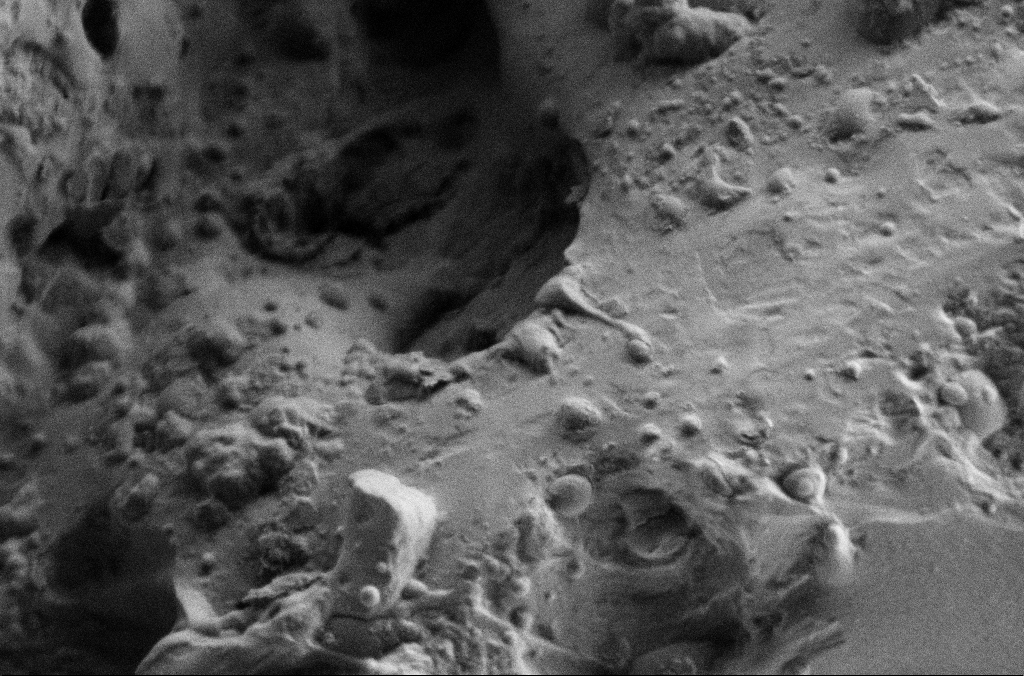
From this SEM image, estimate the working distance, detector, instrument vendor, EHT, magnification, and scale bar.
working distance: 3 mm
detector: SE2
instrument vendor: Zeiss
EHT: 2 kV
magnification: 10 K X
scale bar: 2000 nm